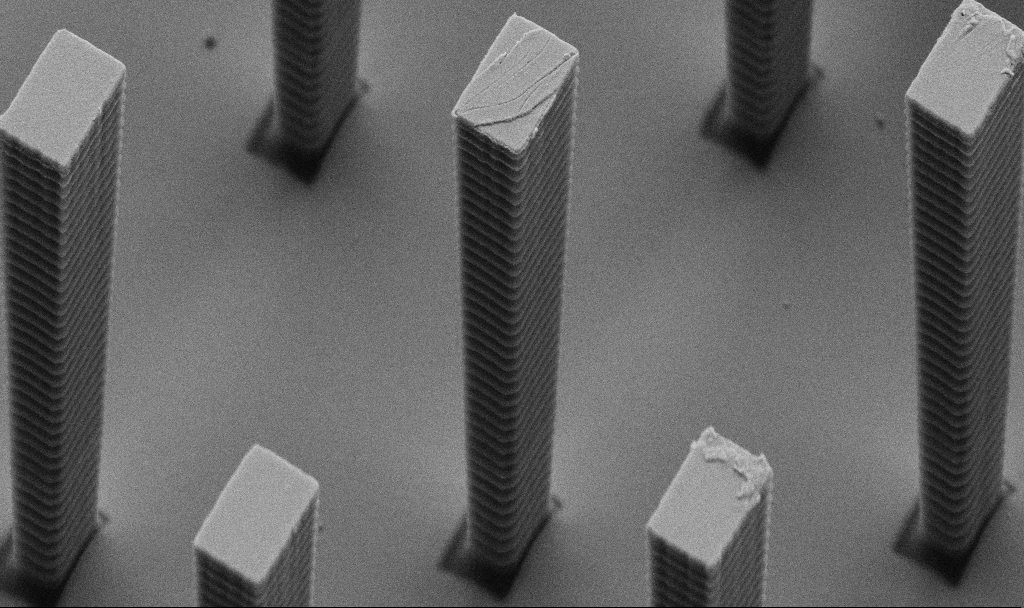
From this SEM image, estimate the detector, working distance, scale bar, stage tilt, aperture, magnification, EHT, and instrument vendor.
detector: SE2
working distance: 5.7 mm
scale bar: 2000 nm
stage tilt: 44.9°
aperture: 30 µm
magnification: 15.33 K X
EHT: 5 kV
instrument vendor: Zeiss